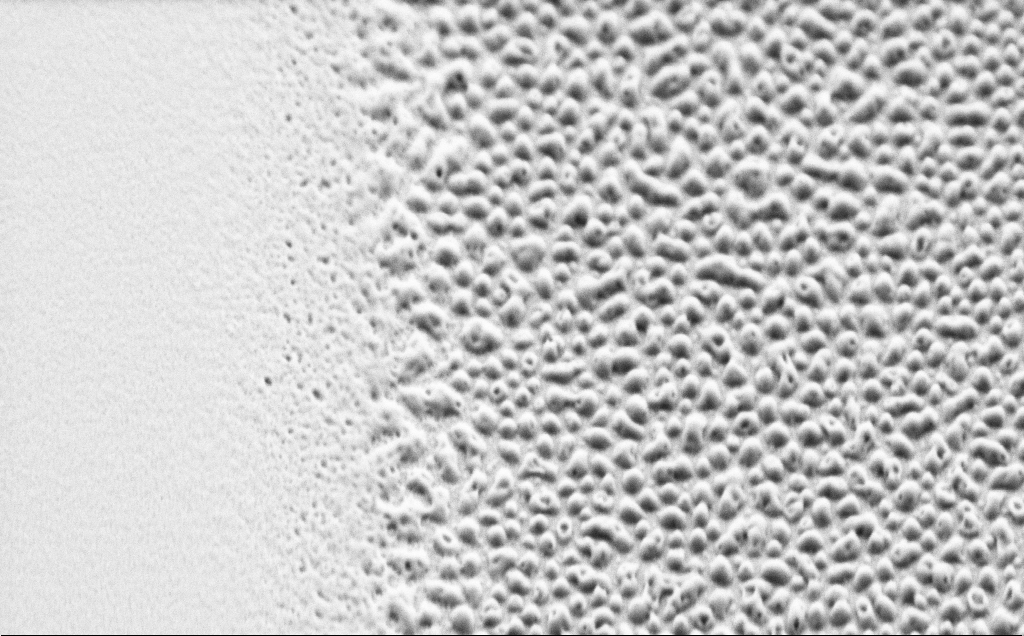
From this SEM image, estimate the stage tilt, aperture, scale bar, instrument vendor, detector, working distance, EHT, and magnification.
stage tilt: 45°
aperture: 30 µm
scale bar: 2000 nm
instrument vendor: Zeiss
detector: SE2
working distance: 4 mm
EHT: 1.5 kV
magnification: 34.8 K X